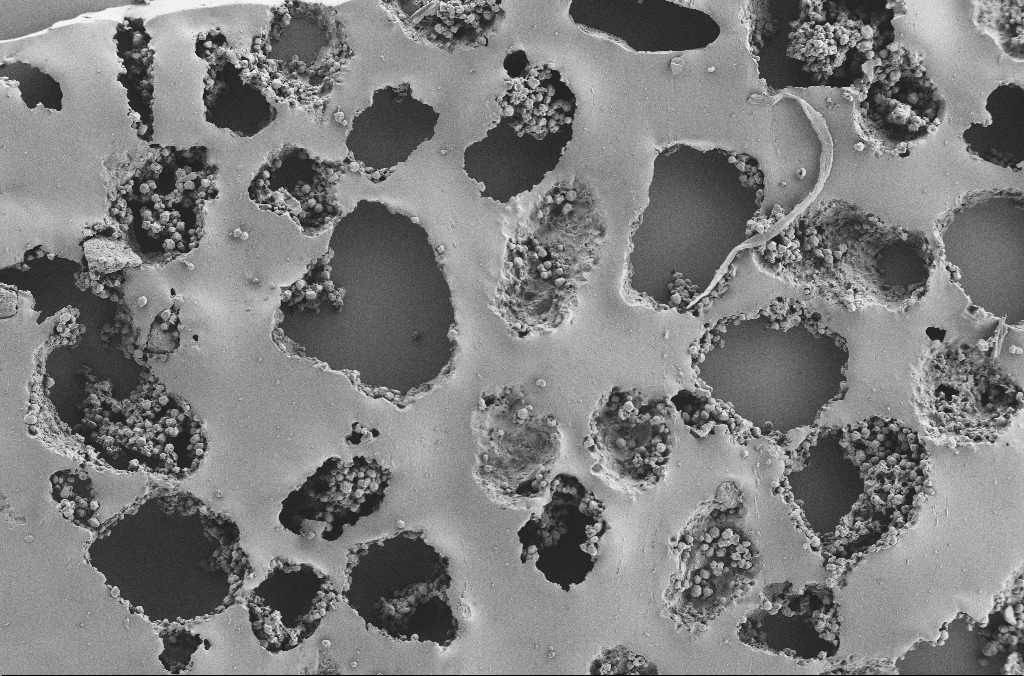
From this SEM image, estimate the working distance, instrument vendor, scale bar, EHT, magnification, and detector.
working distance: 3.7 mm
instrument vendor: Zeiss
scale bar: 100000 nm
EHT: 2 kV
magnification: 0.25 K X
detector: SE2